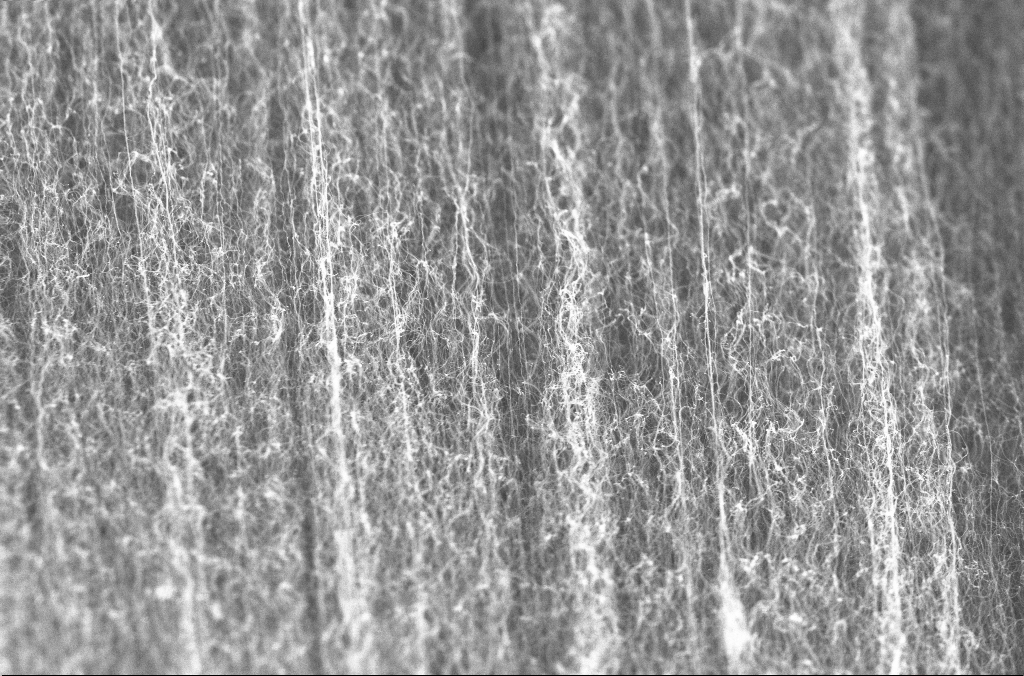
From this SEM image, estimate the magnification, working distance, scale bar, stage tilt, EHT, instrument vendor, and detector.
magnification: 20 K X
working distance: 4 mm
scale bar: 1000 nm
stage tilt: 0°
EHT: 20 kV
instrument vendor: Zeiss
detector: InLens